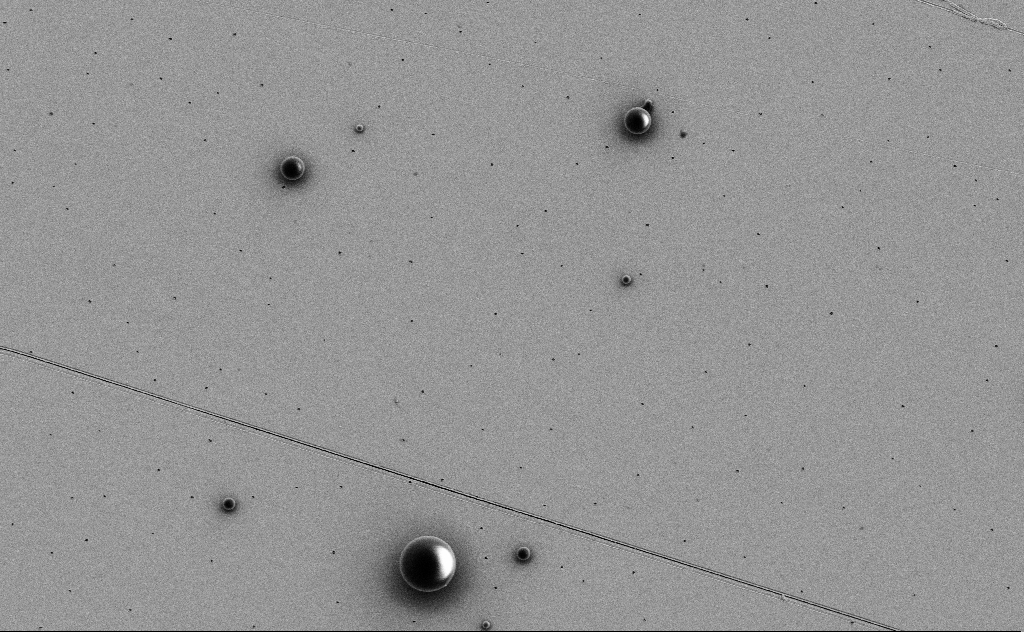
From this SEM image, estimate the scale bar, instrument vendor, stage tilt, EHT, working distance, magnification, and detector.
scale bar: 10000 nm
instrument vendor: Zeiss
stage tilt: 0°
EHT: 3 kV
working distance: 10 mm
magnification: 7.24 K X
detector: SE2